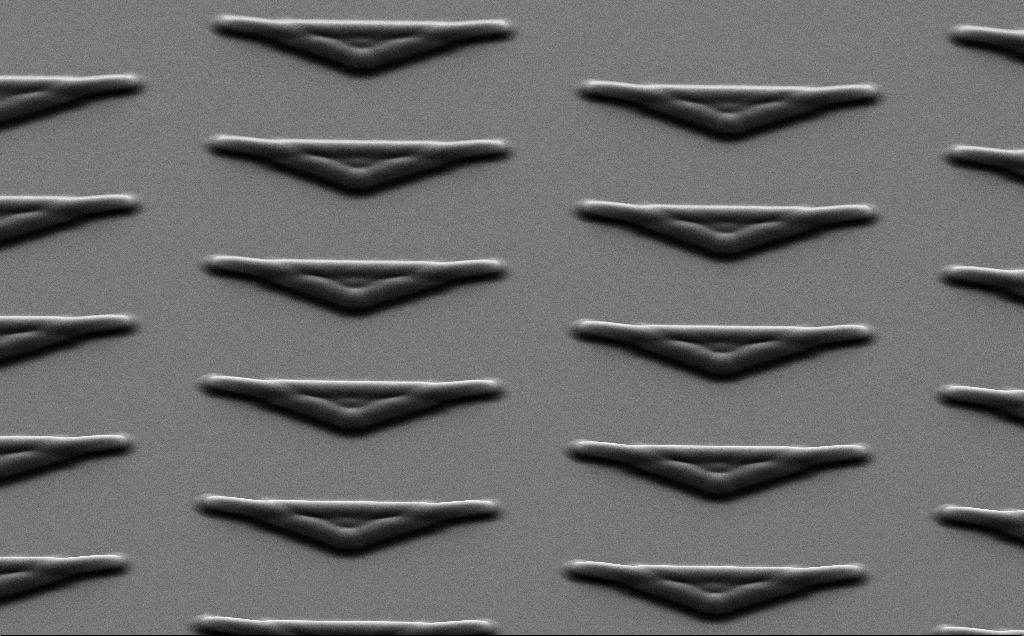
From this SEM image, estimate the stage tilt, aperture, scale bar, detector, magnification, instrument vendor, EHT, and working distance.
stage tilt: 35°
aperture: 30 µm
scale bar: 10000 nm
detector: SE2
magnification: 3.21 K X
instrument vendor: Zeiss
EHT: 7 kV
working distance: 6 mm